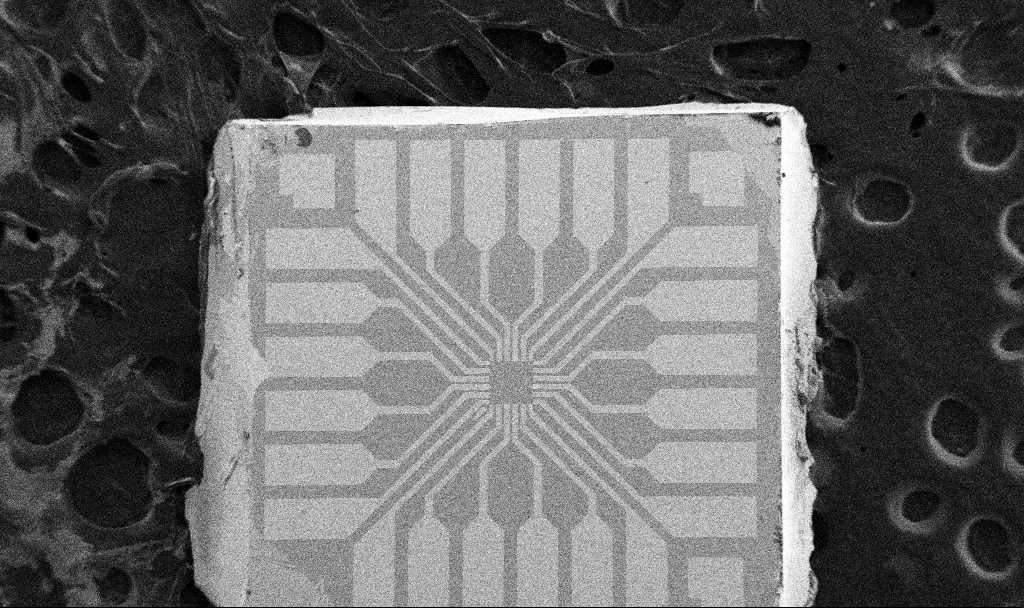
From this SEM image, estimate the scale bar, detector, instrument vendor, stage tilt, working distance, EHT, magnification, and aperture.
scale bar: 200000 nm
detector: SE2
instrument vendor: Zeiss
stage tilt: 0°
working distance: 10.7 mm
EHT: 5 kV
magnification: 0.1 K X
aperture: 30 µm